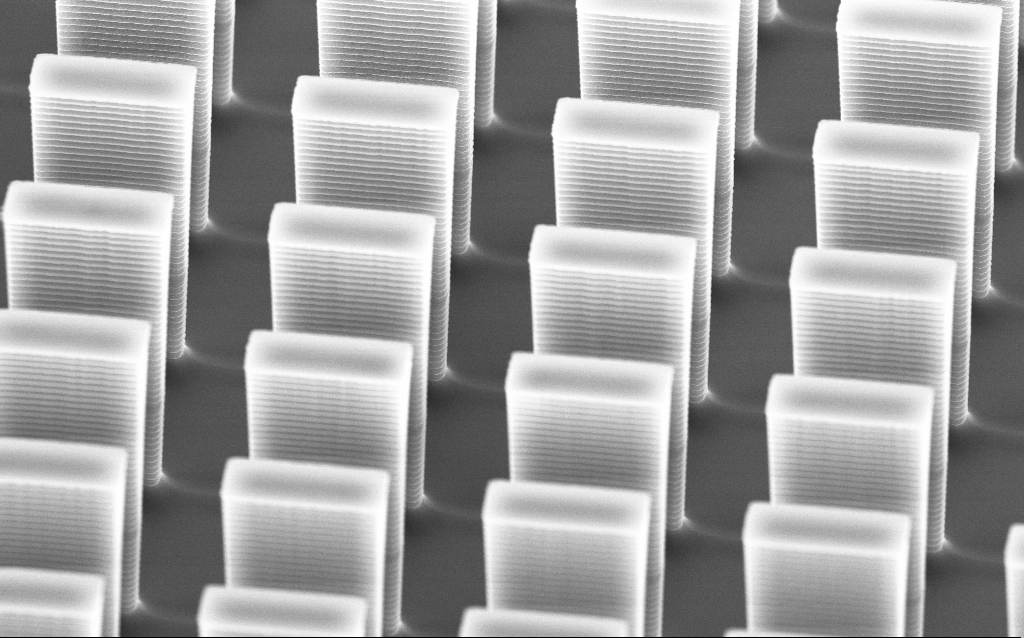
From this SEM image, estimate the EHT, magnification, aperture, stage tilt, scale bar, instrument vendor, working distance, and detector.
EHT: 10 kV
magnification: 12.14 K X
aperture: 30 µm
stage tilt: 45°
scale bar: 2000 nm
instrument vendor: Zeiss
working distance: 5.2 mm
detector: InLens